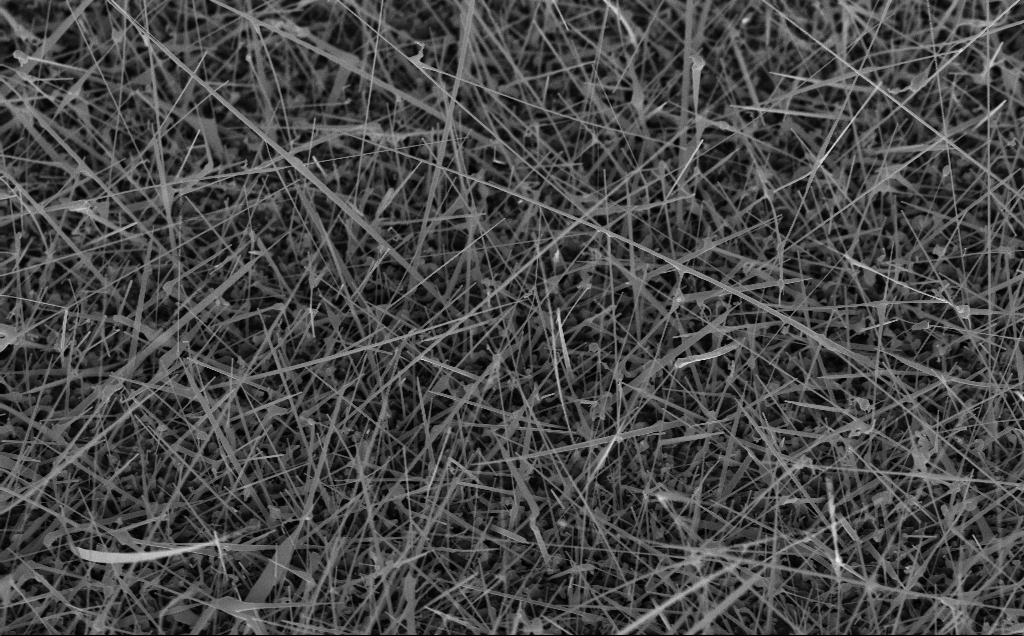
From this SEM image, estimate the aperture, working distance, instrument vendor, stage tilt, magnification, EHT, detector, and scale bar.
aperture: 30 µm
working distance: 8 mm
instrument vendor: Zeiss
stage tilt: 45°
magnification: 19.02 K X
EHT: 10 kV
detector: InLens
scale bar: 2000 nm